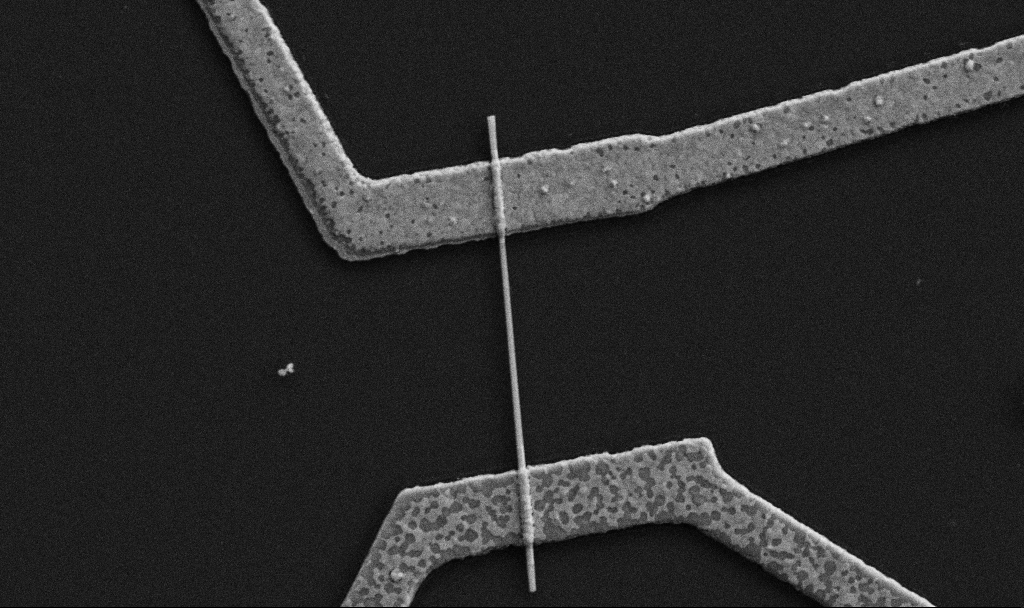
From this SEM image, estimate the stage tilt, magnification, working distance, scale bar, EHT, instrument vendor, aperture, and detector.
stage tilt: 0°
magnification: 30 K X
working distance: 8.7 mm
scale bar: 1000 nm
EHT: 5 kV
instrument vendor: Zeiss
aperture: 30 µm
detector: SE2